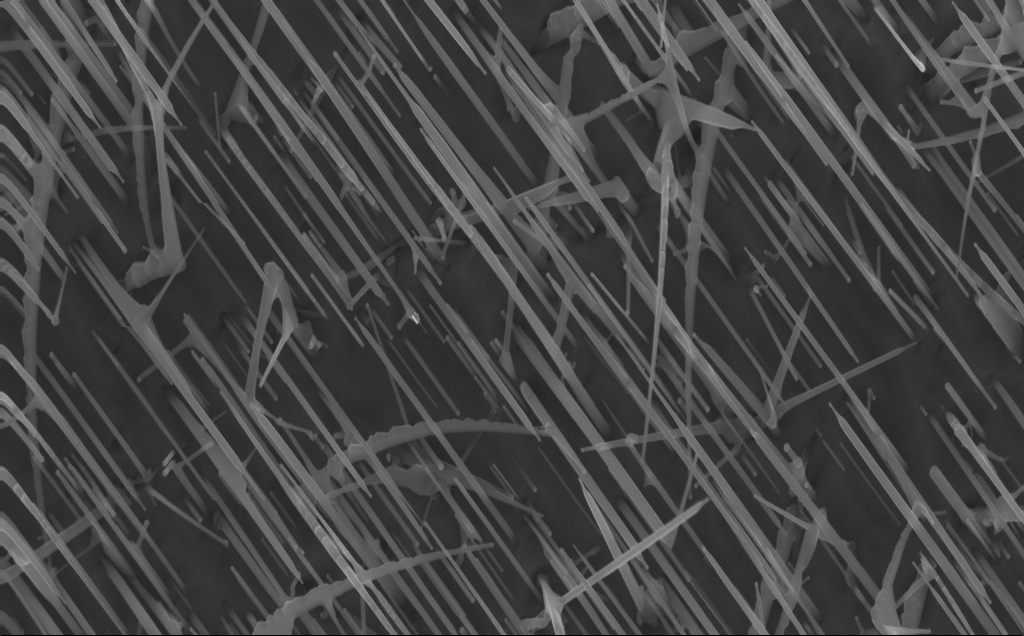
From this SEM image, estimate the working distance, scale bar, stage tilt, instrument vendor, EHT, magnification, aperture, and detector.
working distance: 4 mm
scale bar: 1000 nm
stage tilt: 0°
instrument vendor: Zeiss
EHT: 10 kV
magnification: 40 K X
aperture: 30 µm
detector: InLens